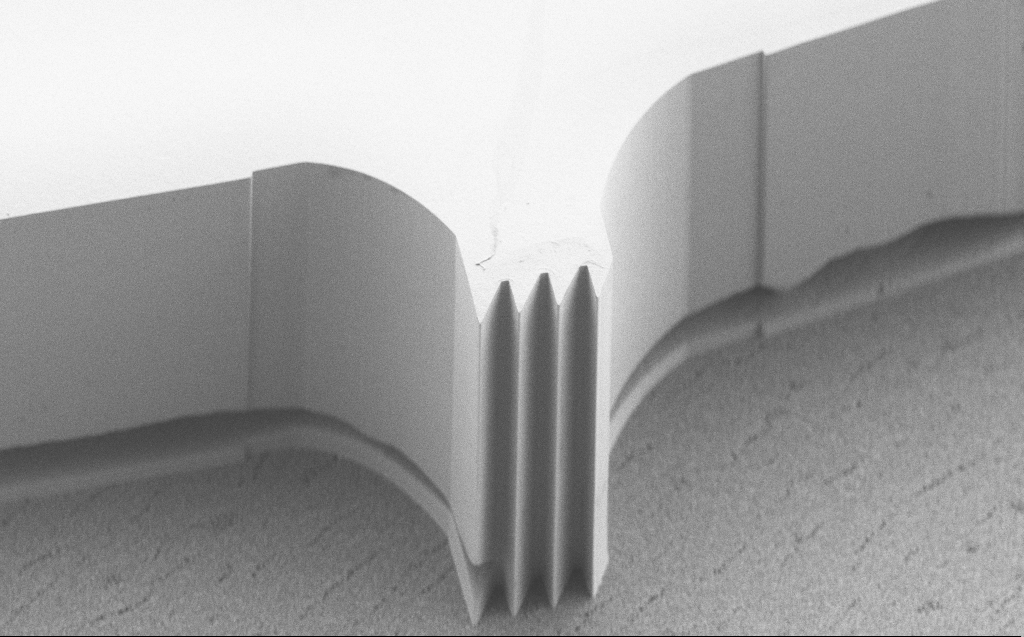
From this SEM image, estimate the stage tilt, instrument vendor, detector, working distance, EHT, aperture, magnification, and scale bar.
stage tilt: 45°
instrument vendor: Zeiss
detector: SE2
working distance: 5 mm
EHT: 5 kV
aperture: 30 µm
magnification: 1.33 K X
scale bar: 10000 nm